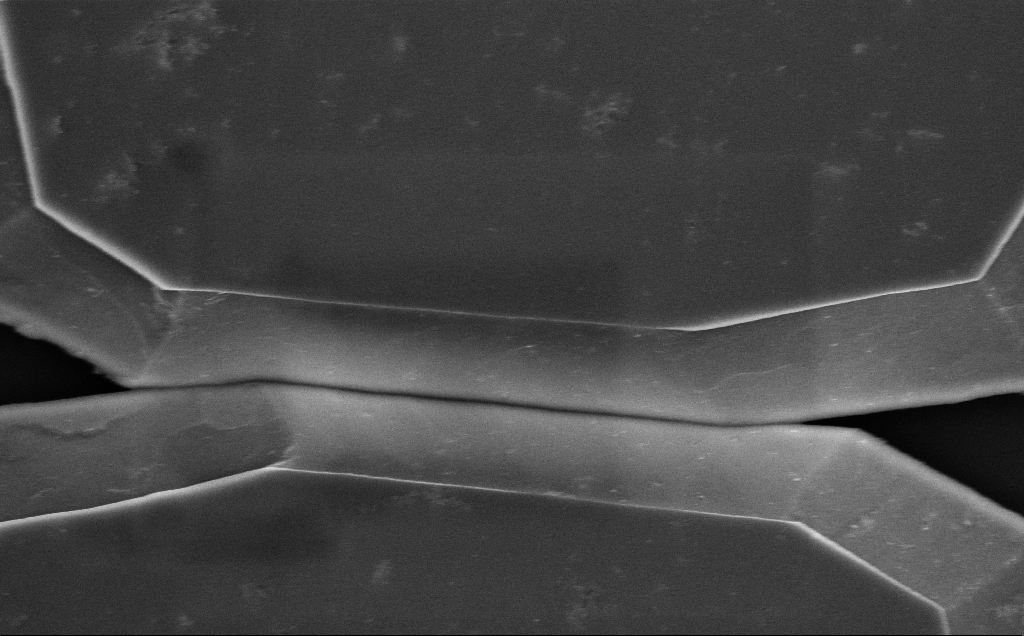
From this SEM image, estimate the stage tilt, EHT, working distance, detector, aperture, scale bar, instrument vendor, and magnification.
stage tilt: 0°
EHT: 5 kV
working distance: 14 mm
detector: InLens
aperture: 30 µm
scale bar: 1000 nm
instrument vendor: Zeiss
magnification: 20.92 K X